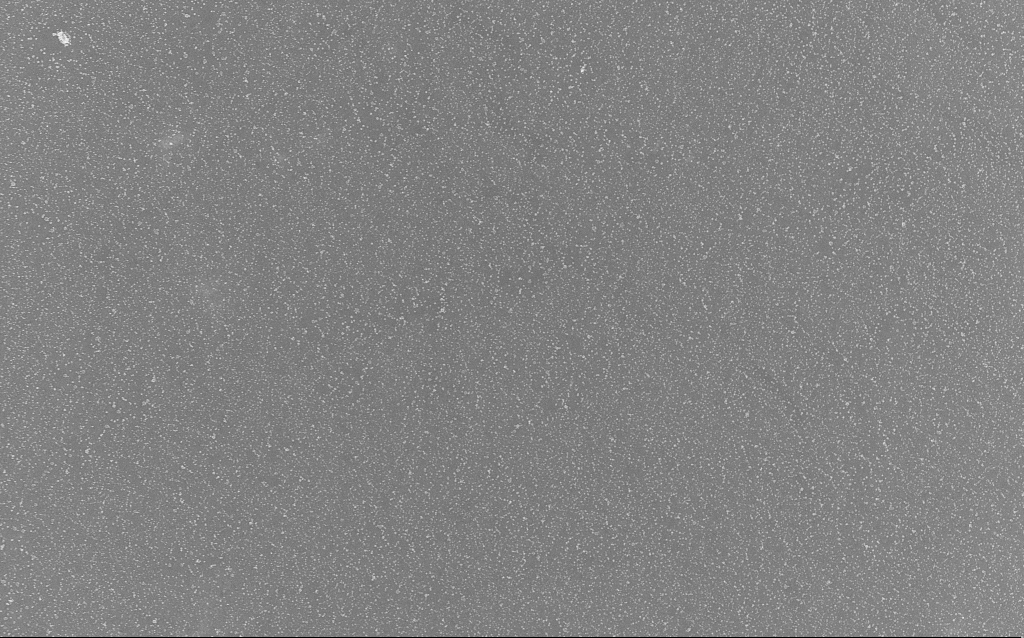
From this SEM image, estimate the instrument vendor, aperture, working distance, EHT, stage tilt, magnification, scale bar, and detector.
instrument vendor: Zeiss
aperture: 30 µm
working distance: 1.5 mm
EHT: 20 kV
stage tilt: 0°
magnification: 1 K X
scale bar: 20000 nm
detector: InLens